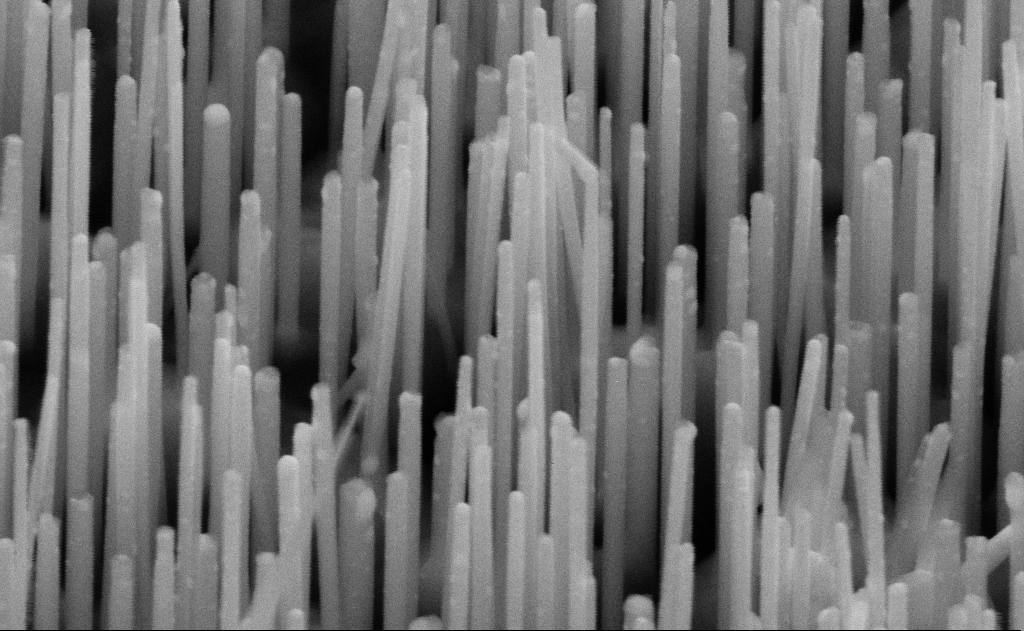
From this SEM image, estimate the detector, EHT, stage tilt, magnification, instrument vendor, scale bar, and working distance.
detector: SE2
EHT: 10 kV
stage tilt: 45°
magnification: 150 K X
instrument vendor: Zeiss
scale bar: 200 nm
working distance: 11 mm